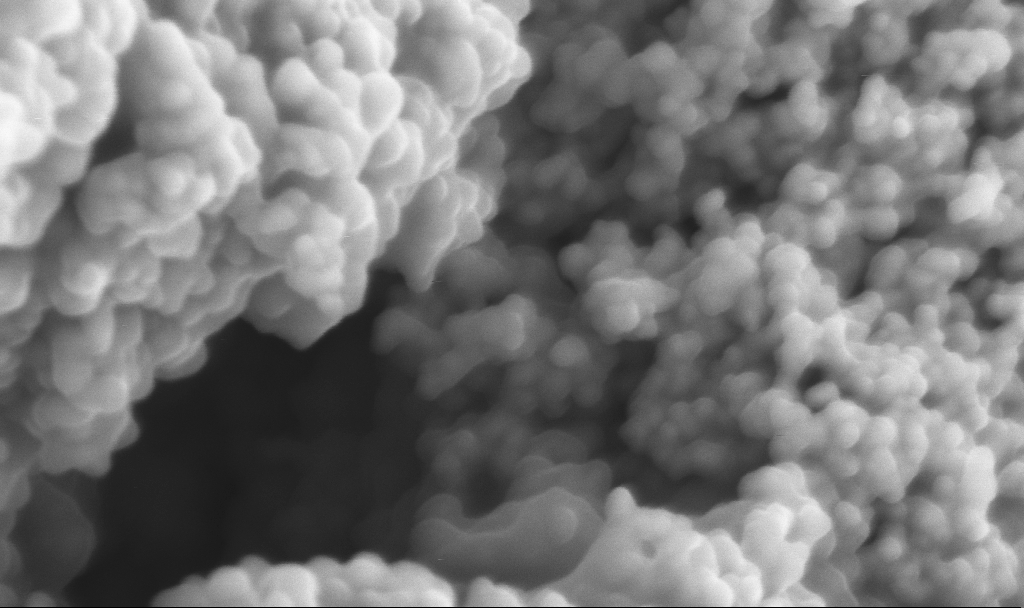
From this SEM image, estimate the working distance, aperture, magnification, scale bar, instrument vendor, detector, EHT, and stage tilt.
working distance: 2.5 mm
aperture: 30 µm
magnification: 268.23 K X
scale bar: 200 nm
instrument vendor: Zeiss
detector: InLens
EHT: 3 kV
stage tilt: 0°